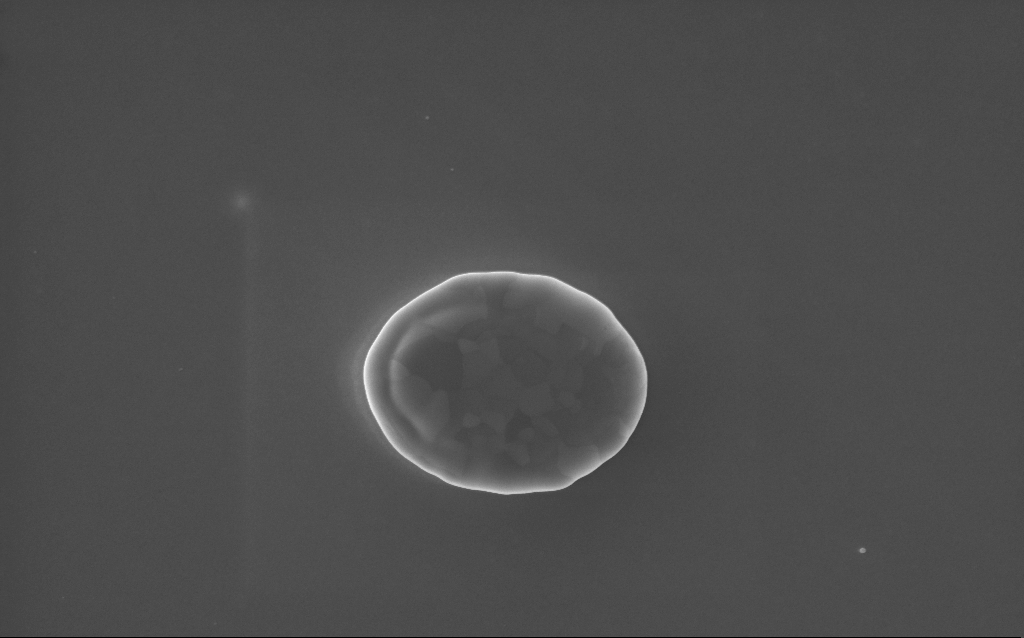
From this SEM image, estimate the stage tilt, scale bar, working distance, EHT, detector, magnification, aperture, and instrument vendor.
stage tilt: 0°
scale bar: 2000 nm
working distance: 2 mm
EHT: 10 kV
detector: InLens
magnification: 35.24 K X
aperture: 30 µm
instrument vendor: Zeiss